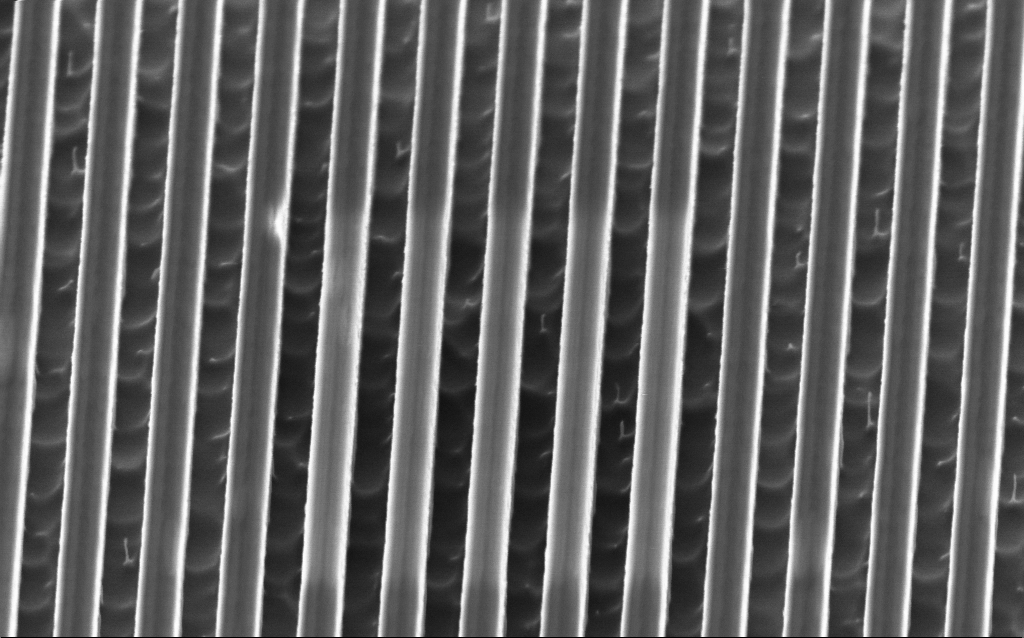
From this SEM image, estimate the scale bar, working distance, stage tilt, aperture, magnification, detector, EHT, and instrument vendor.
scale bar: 1000 nm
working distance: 3.3 mm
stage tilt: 45°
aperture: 30 µm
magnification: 59.97 K X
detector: InLens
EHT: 2 kV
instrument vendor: Zeiss